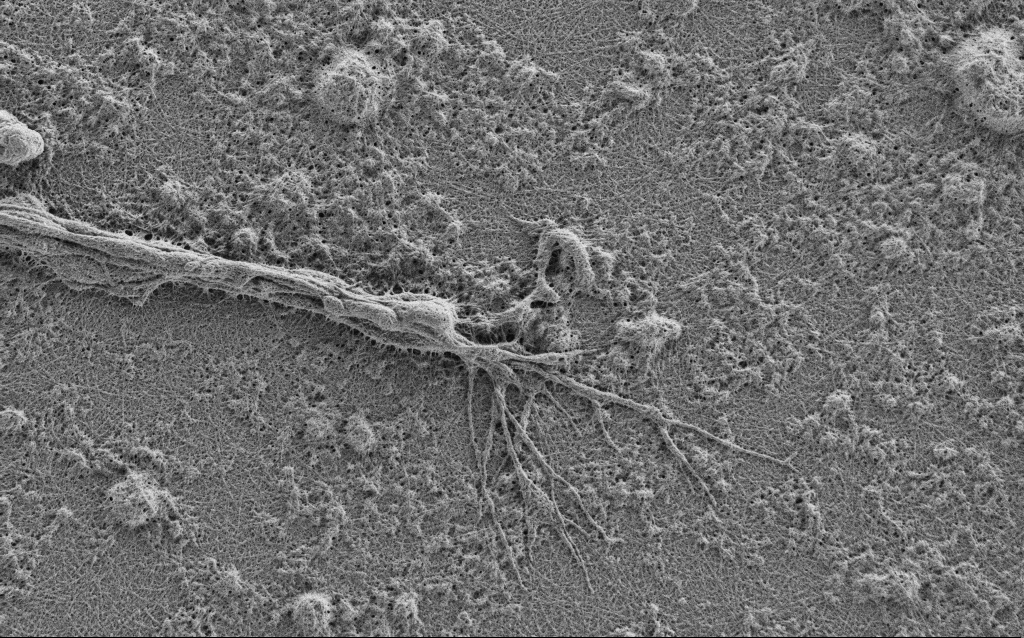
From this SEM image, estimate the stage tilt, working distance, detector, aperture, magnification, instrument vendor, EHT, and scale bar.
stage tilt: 0°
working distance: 4 mm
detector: SE2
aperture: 30 µm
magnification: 10 K X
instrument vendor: Zeiss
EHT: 0.9 kV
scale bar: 2000 nm